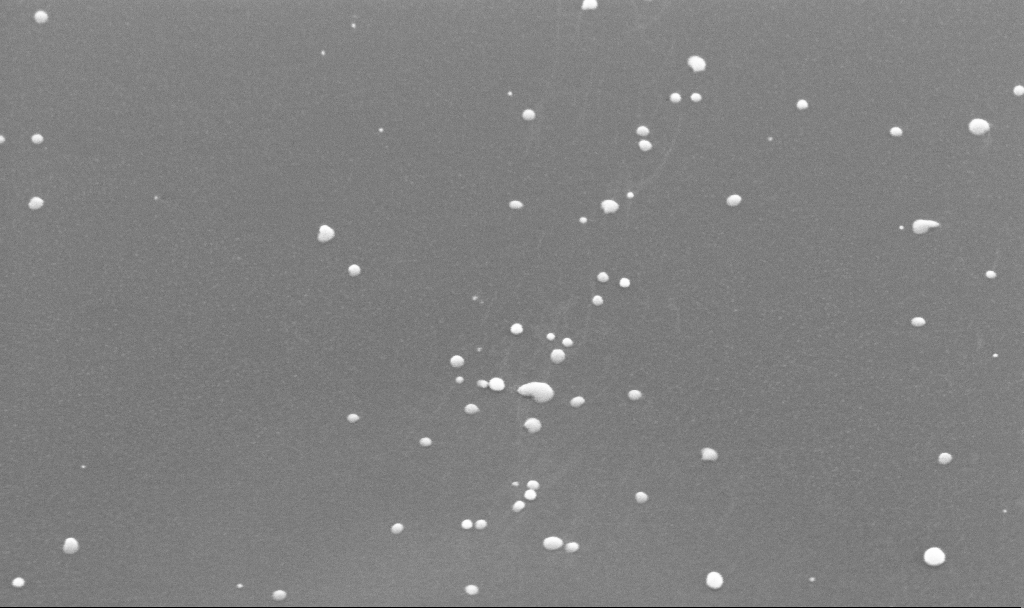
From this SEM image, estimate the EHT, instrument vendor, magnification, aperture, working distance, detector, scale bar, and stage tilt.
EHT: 10 kV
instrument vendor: Zeiss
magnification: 150 K X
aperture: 30 µm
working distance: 4 mm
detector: InLens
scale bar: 100 nm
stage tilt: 45°